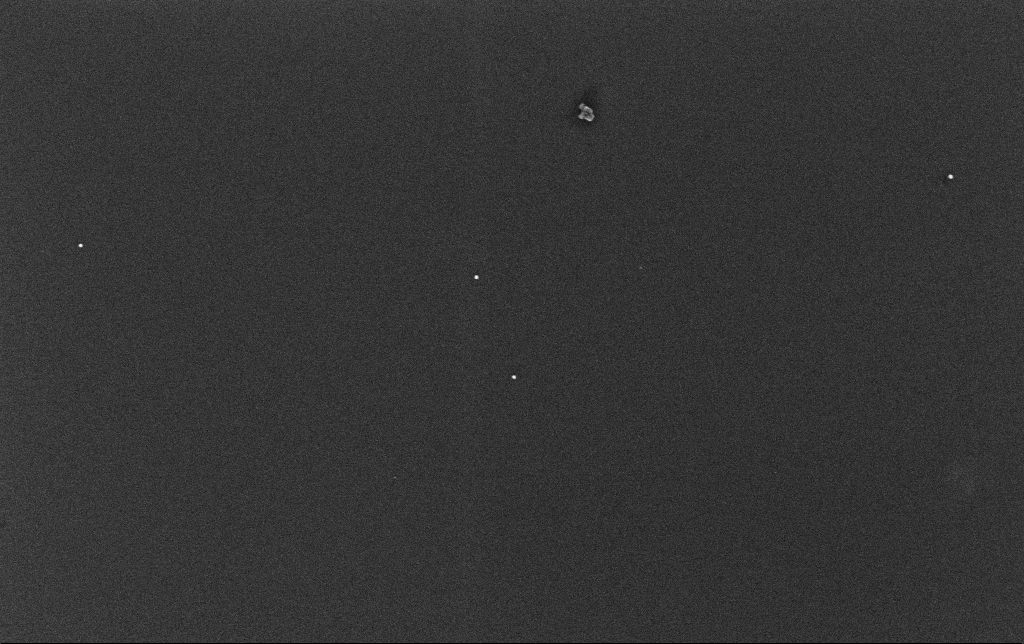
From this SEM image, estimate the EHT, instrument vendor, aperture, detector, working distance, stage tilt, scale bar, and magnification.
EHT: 10 kV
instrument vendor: Zeiss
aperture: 30 µm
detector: InLens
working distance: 3.4 mm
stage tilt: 0°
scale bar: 200 nm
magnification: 100 K X